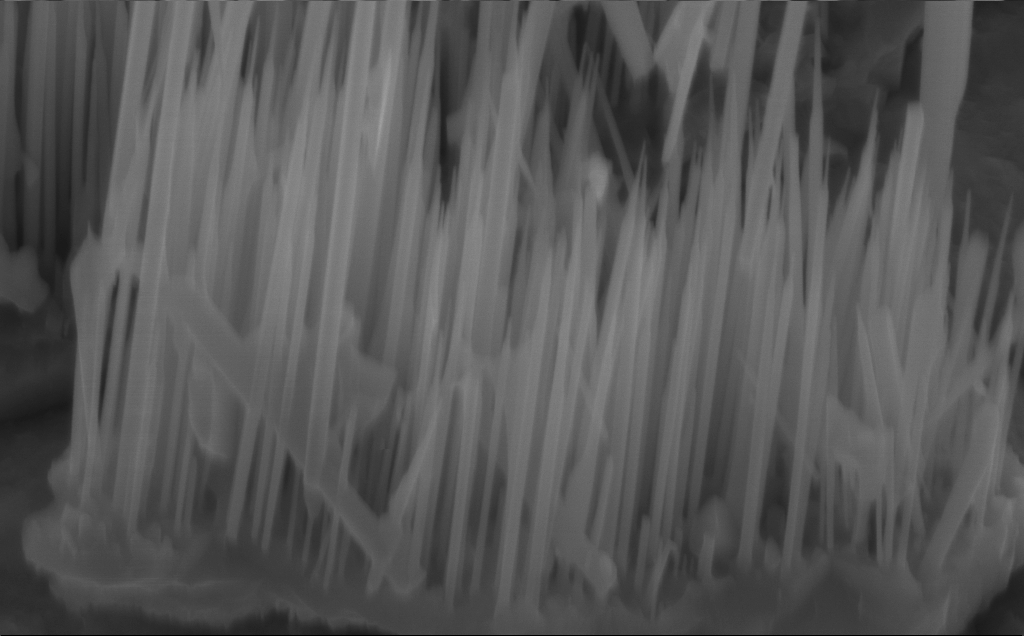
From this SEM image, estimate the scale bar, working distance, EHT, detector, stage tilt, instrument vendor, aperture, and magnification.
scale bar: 1000 nm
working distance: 5 mm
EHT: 10 kV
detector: InLens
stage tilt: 45°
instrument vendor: Zeiss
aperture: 30 µm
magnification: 65.43 K X